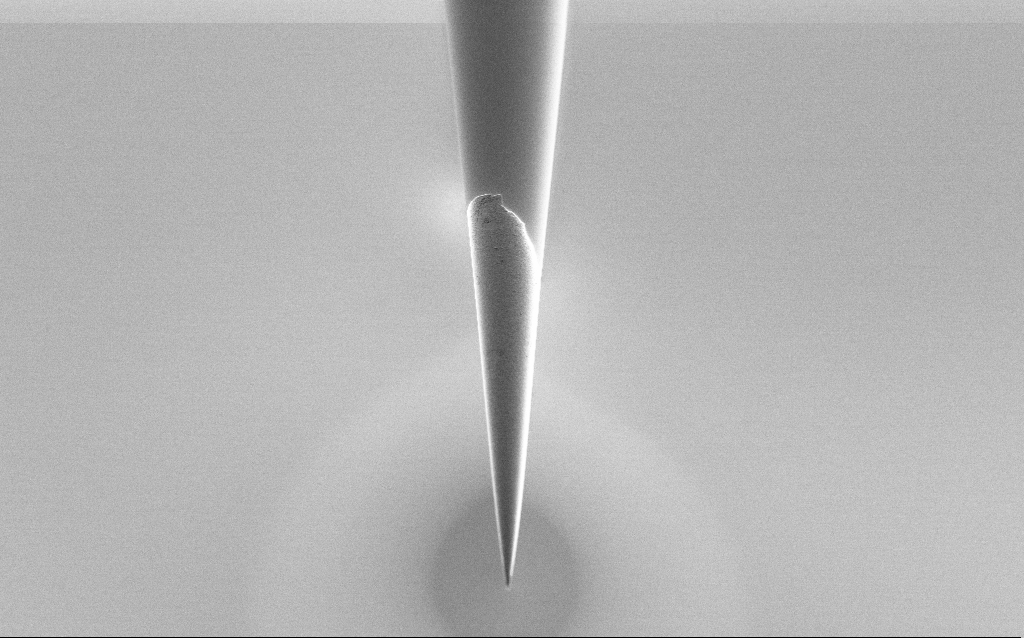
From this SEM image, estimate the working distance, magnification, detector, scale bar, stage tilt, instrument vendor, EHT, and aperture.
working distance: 6 mm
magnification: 5 K X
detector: SE2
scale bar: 10000 nm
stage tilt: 45°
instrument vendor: Zeiss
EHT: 1 kV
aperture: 30 µm